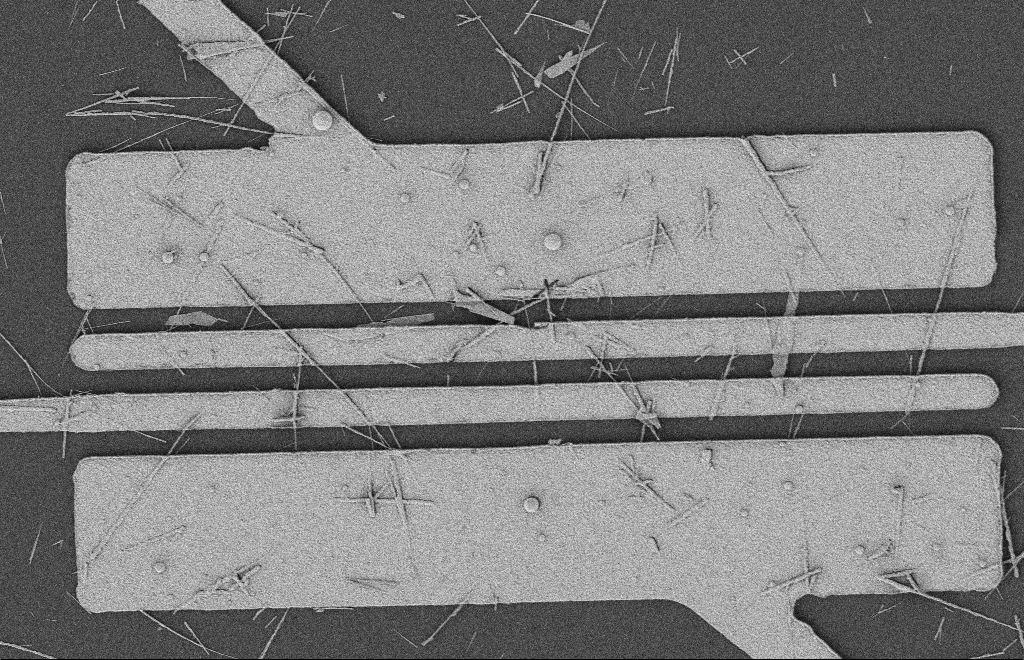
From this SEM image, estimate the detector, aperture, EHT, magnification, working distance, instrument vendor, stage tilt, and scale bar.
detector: SE2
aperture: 20 µm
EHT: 2 kV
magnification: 5.54 K X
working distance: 12 mm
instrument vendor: Zeiss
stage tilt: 0°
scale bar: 2000 nm